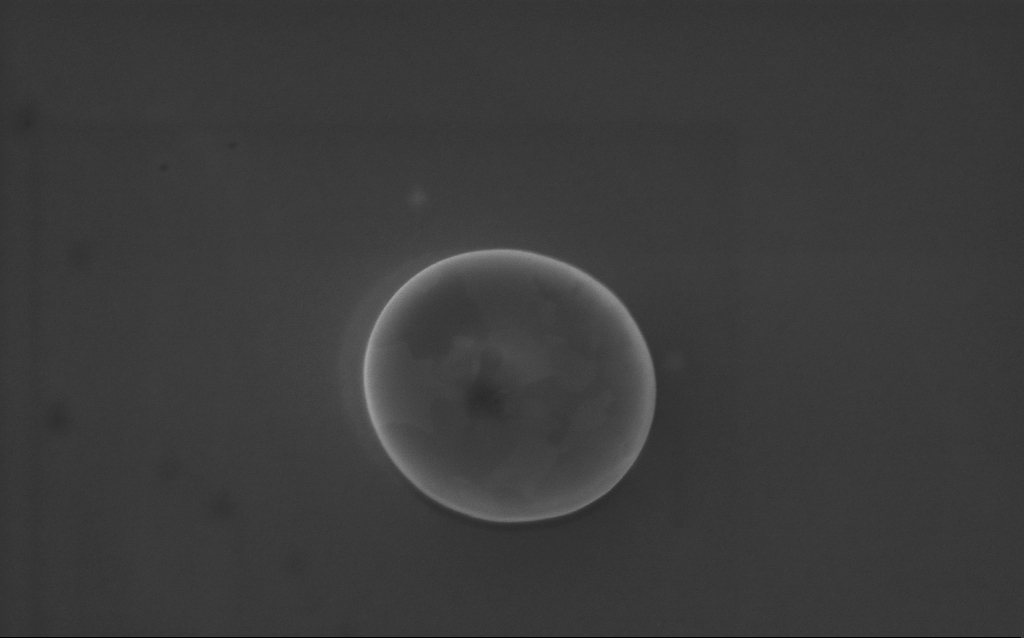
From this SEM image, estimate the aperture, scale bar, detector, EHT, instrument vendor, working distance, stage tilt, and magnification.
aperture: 30 µm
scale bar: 1000 nm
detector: InLens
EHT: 5 kV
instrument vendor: Zeiss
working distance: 3 mm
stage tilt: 0°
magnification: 62.16 K X